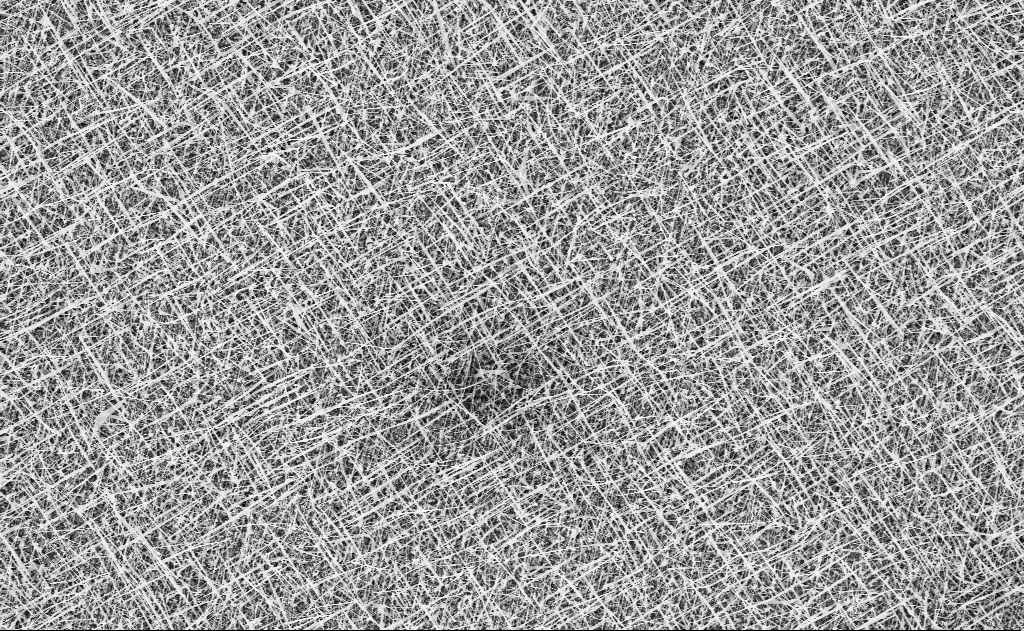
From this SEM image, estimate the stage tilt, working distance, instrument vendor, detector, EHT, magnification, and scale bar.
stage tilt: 0°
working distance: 20 mm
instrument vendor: Zeiss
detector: InLens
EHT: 10 kV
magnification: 5 K X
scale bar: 10000 nm